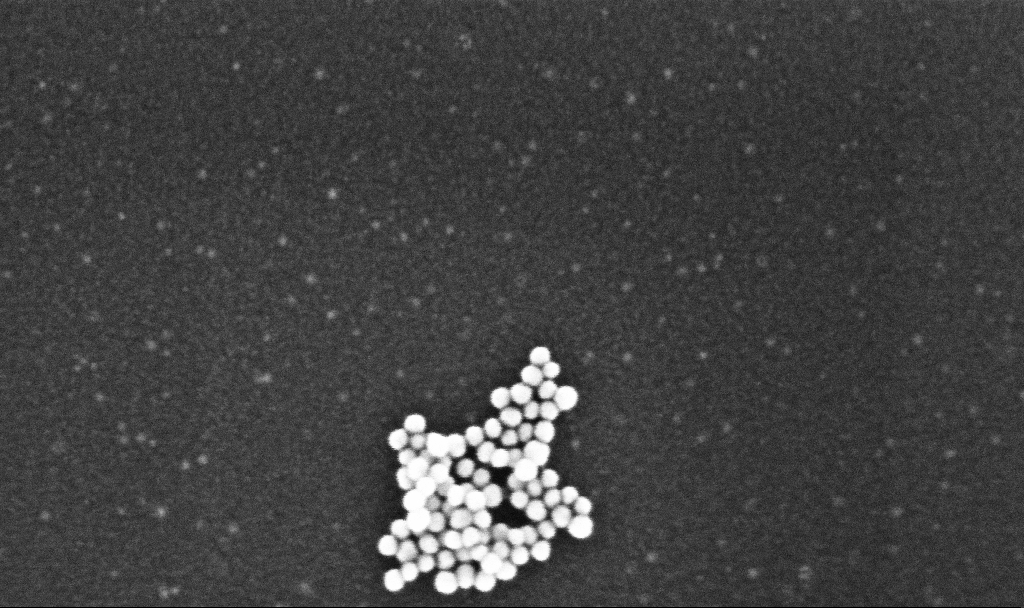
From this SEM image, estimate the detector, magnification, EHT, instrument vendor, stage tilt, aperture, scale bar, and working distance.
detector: InLens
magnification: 333.51 K X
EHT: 10 kV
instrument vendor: Zeiss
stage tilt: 0°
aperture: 30 µm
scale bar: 200 nm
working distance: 3.1 mm